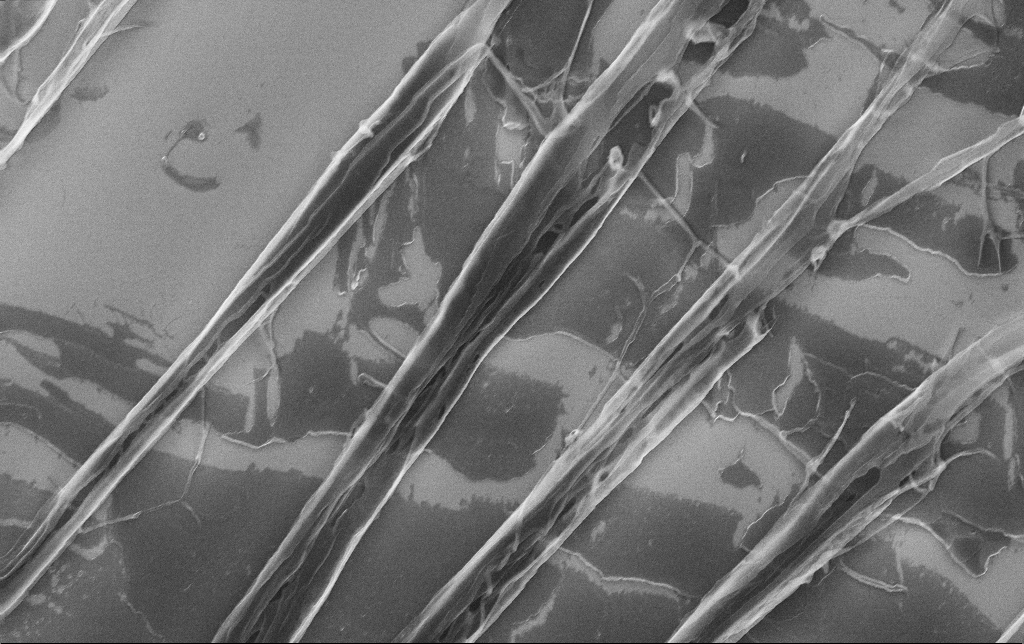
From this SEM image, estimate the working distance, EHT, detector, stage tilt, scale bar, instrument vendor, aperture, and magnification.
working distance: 3.1 mm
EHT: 10 kV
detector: InLens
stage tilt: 0°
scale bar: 1000 nm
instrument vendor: Zeiss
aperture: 30 µm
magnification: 16.19 K X